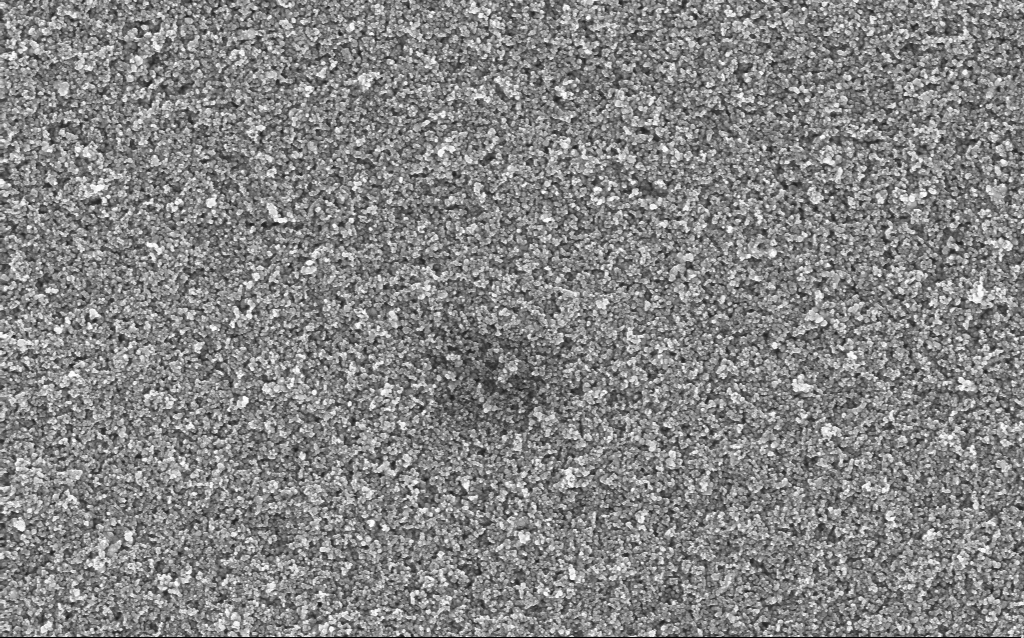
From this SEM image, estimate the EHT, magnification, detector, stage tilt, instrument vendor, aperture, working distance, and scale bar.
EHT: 5 kV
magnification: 37.88 K X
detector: InLens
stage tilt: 0°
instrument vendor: Zeiss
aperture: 30 µm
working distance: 6.4 mm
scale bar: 1000 nm